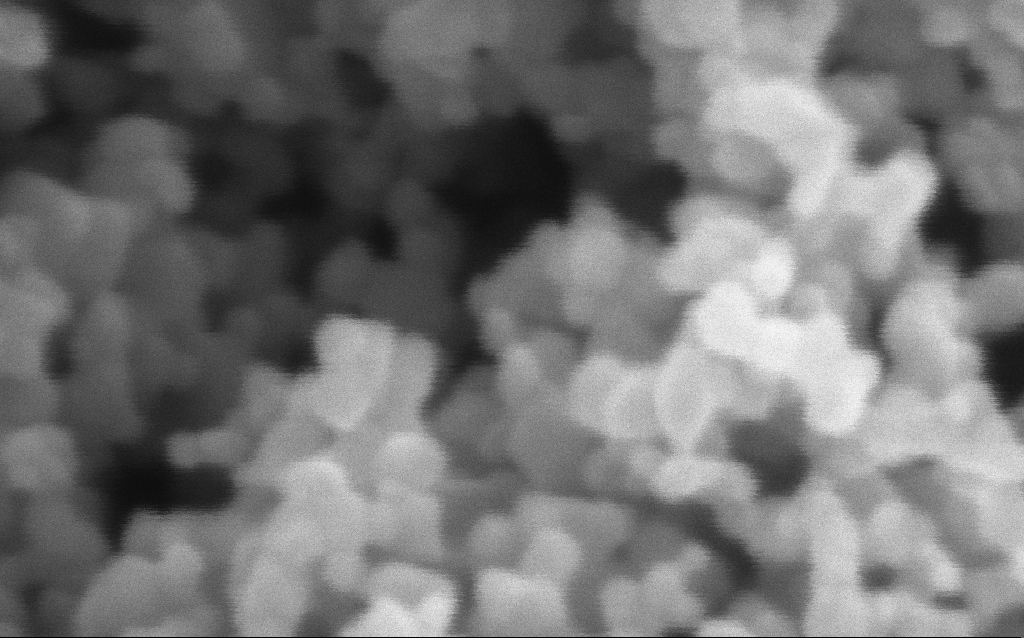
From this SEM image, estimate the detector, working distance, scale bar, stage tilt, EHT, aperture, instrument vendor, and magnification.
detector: InLens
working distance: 7.5 mm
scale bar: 100 nm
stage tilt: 0°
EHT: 3 kV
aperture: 30 µm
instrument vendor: Zeiss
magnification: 716 K X